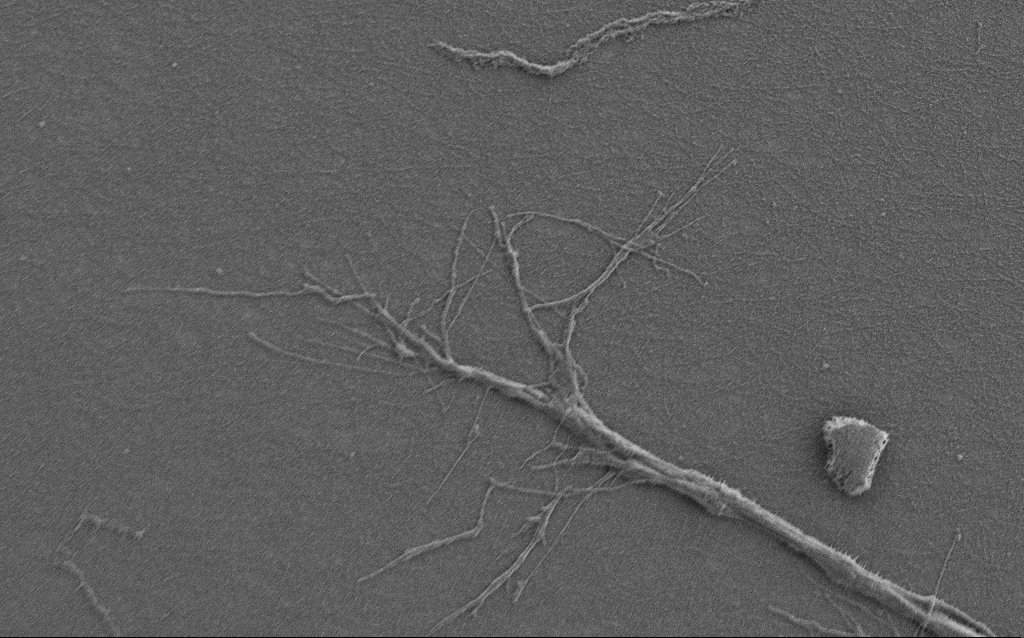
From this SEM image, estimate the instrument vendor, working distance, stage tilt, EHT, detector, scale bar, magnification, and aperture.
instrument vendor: Zeiss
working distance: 6 mm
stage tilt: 0°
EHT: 0.9 kV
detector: SE2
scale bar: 10000 nm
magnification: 6.5 K X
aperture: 30 µm